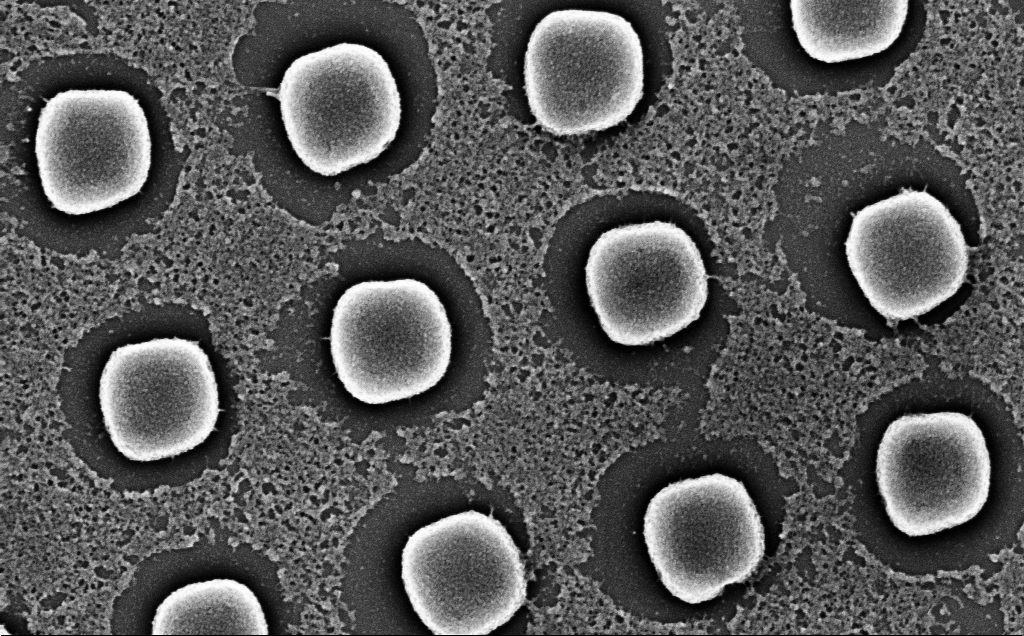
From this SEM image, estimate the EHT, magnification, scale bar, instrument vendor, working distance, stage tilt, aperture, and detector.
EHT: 5 kV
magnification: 61.82 K X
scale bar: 1000 nm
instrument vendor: Zeiss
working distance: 7 mm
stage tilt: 0°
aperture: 30 µm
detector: InLens